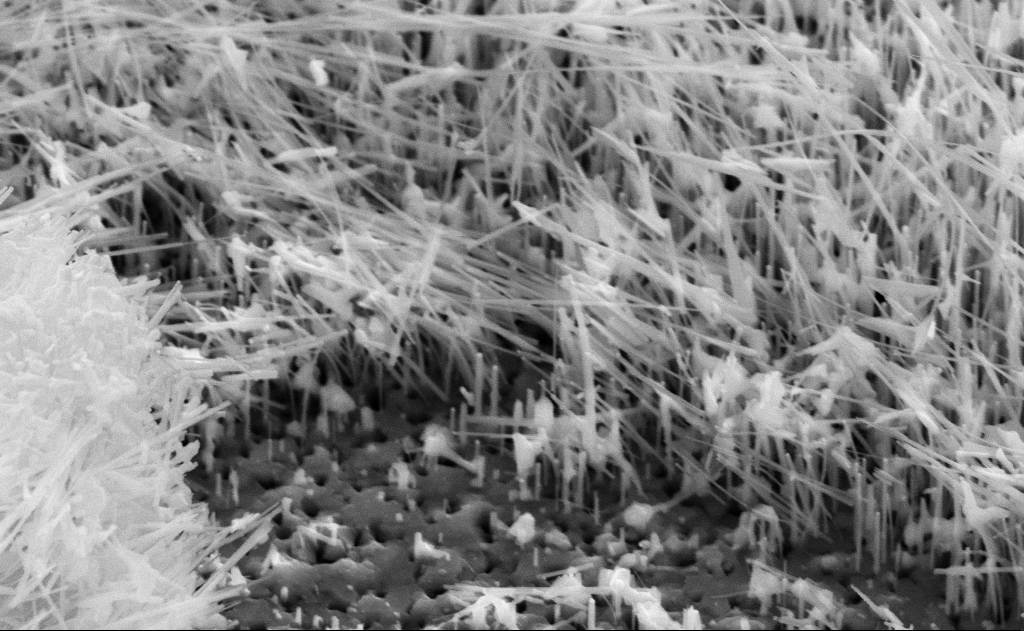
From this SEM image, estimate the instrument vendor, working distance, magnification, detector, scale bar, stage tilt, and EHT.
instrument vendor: Zeiss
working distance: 14 mm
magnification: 40 K X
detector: SE2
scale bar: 1000 nm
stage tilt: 45°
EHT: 10 kV